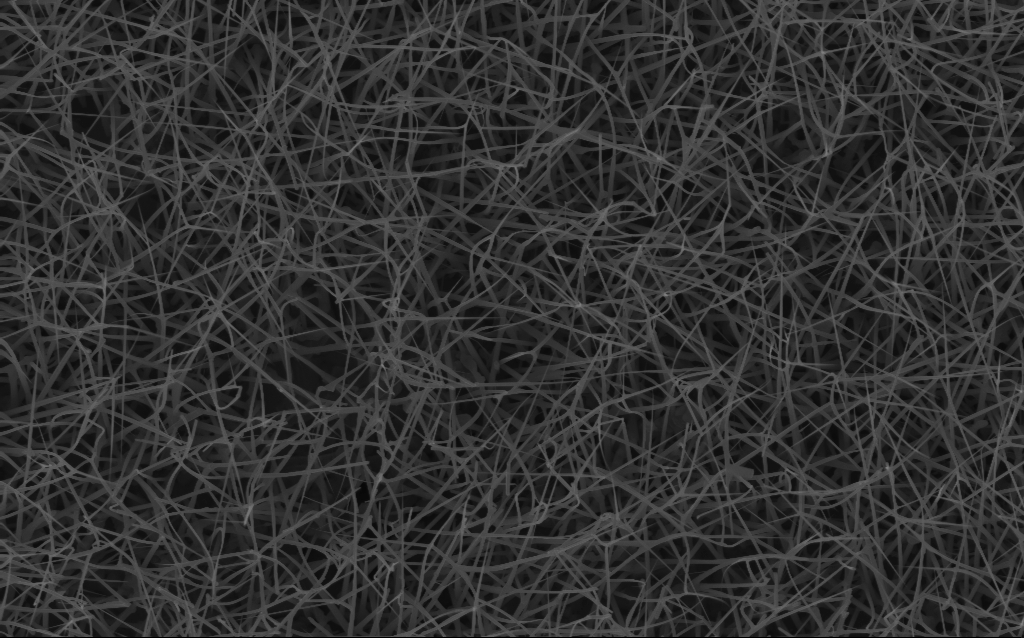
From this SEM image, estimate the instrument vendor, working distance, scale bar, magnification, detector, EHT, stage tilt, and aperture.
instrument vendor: Zeiss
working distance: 6 mm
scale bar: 2000 nm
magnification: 10 K X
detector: InLens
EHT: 10 kV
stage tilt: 0°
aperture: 30 µm